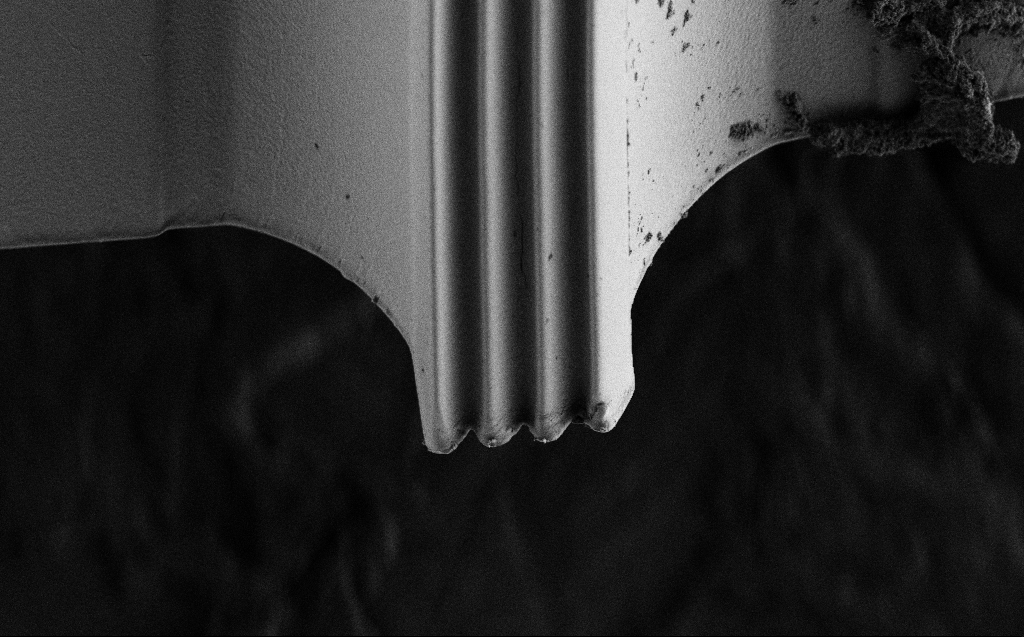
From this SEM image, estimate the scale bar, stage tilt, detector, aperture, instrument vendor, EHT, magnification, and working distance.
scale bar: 10000 nm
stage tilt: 44.6°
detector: SE2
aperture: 30 µm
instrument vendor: Zeiss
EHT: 1 kV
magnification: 1.76 K X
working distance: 6 mm